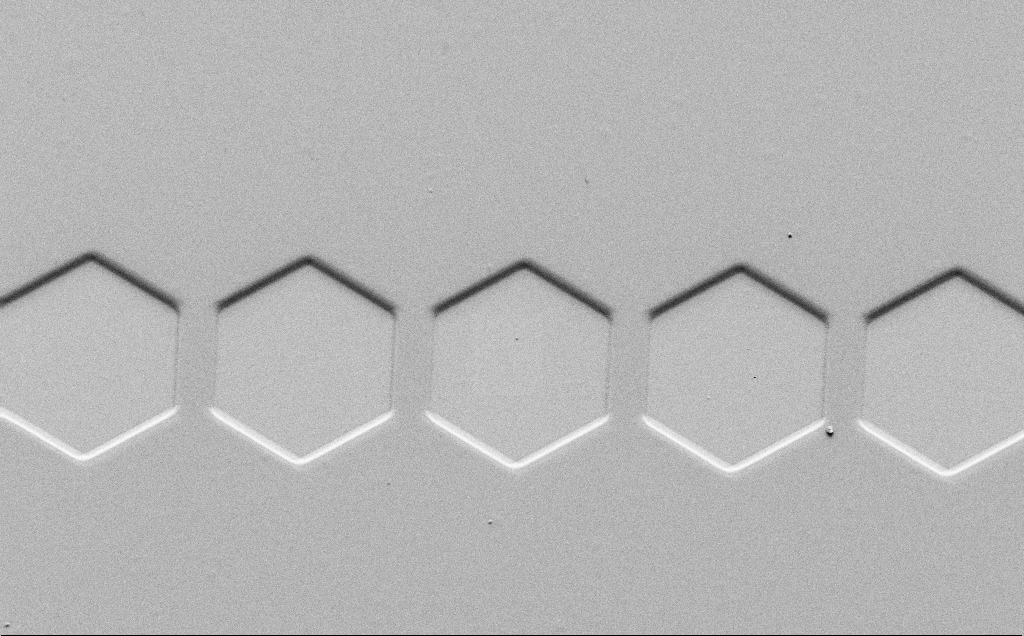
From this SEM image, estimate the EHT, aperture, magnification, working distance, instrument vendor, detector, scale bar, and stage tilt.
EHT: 1.5 kV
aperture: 30 µm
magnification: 1.35 K X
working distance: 4 mm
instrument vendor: Zeiss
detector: SE2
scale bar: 10000 nm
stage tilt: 45°